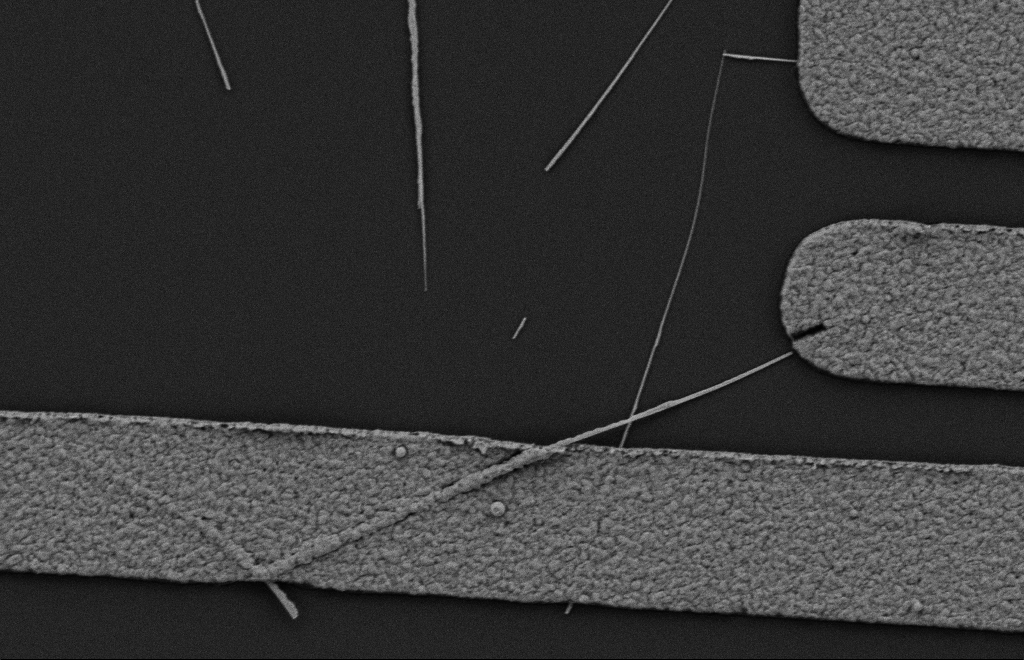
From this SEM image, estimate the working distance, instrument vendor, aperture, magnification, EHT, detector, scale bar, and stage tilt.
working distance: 10 mm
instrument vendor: Zeiss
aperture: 20 µm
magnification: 21.93 K X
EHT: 2 kV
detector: SE2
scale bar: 2000 nm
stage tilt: -0.3°